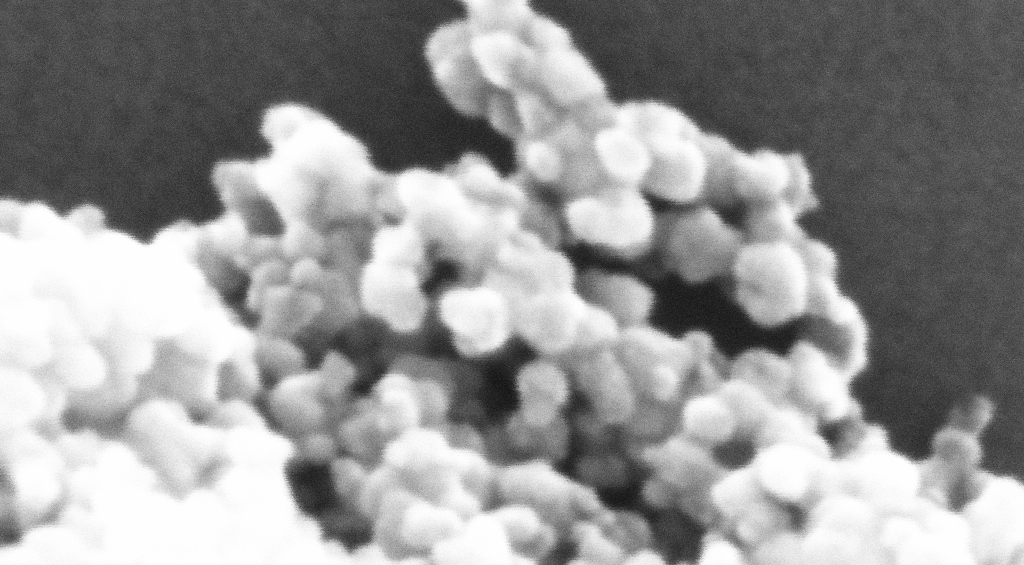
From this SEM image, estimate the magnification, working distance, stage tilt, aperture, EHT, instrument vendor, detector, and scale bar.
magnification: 993.27 K X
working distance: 5.2 mm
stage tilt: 0°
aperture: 30 µm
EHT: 10 kV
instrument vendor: Zeiss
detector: InLens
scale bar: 20 nm